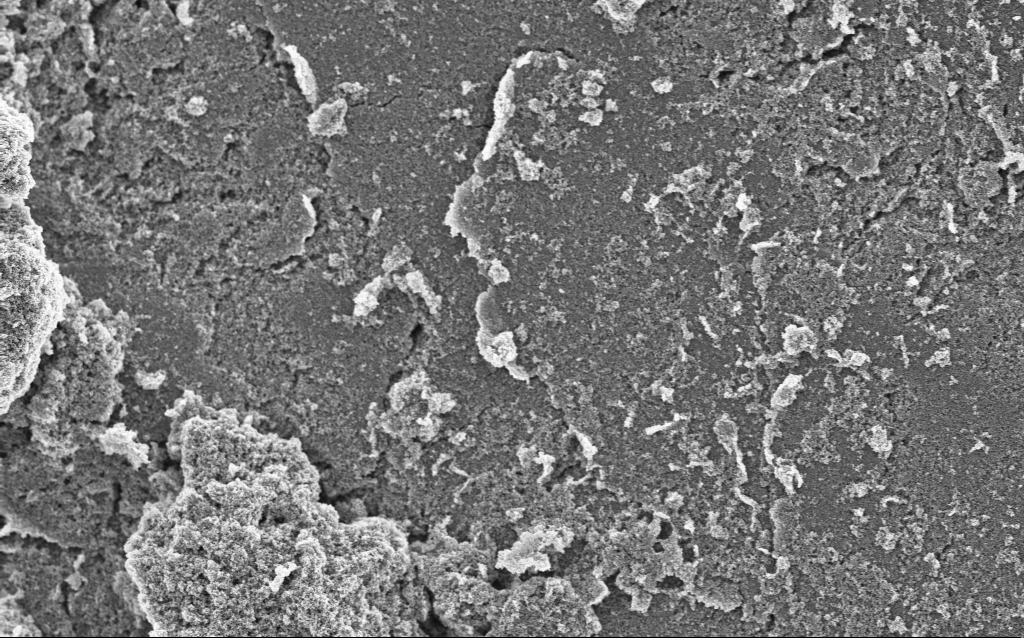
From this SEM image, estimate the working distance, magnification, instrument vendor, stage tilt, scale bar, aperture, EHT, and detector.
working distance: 4.4 mm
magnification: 6.42 K X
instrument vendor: Zeiss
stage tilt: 0°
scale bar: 10000 nm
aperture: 30 µm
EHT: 5 kV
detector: InLens